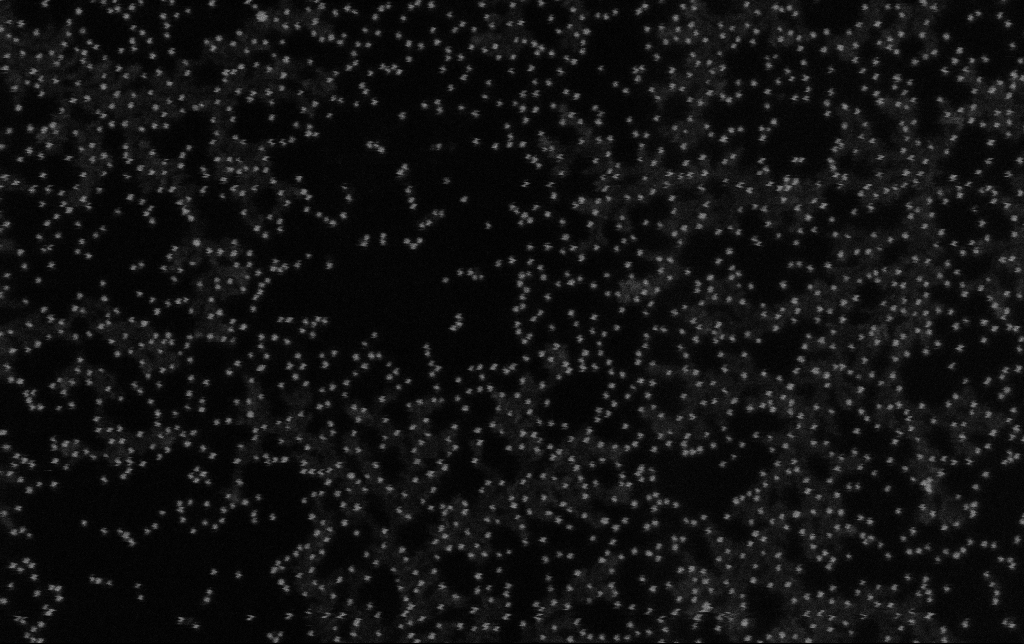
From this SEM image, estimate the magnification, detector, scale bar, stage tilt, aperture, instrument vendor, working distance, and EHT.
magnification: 175.72 K X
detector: SE2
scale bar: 100 nm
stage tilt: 0°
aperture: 30 µm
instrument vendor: Zeiss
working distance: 11.3 mm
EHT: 10 kV